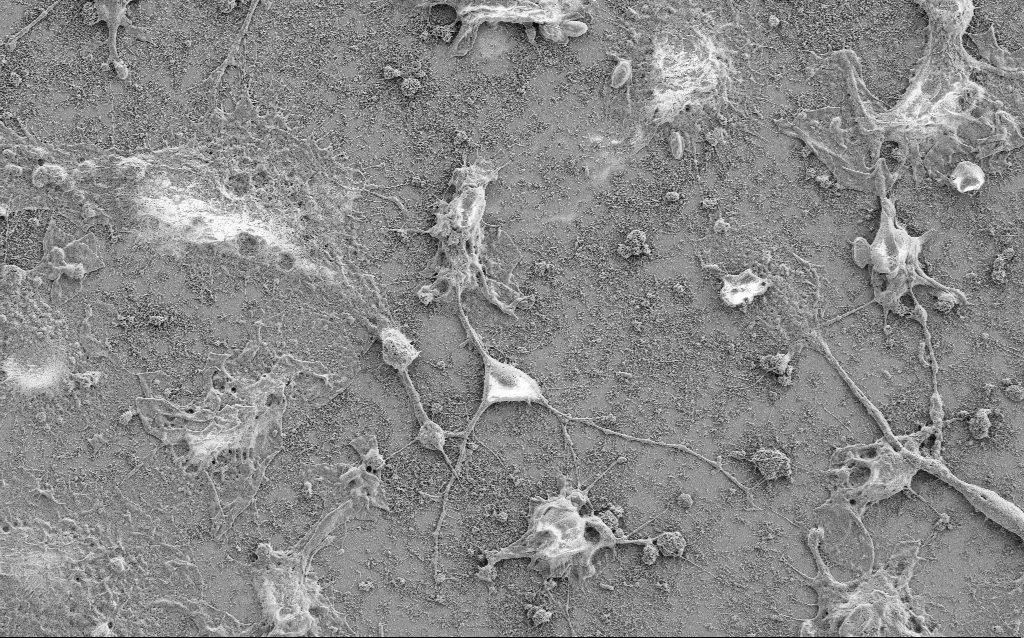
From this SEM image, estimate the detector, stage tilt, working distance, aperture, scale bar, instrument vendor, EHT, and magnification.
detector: SE2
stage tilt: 0°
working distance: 6.8 mm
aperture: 30 µm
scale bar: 10000 nm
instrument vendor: Zeiss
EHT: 2 kV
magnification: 2.5 K X